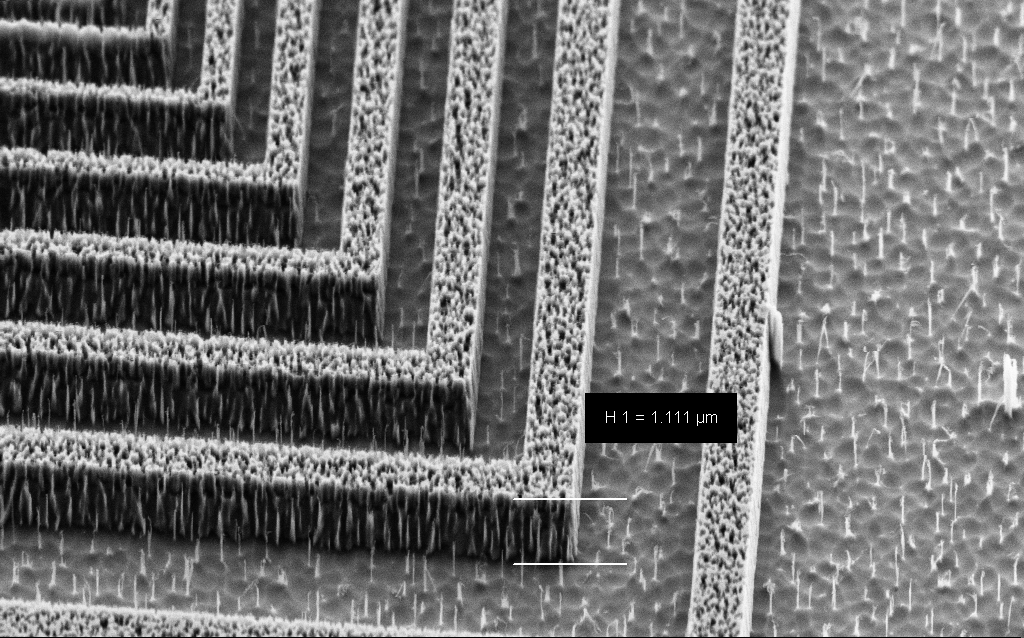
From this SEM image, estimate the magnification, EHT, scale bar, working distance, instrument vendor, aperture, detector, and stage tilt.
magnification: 21.49 K X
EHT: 3 kV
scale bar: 2000 nm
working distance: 8 mm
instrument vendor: Zeiss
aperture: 30 µm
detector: SE2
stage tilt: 45°